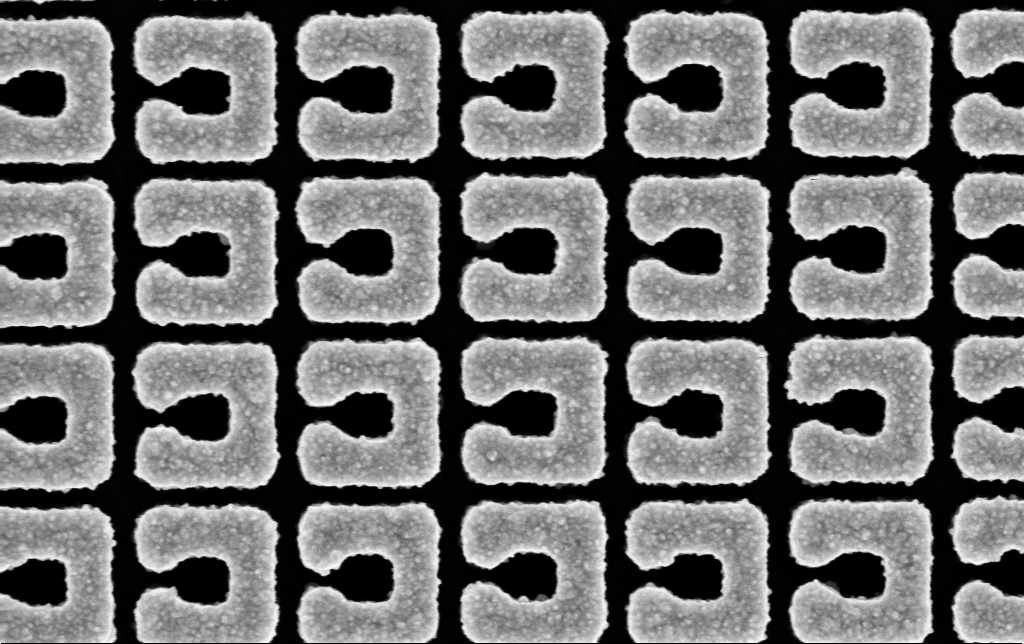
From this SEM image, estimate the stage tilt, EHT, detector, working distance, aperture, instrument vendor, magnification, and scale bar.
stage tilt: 0°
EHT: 5 kV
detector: InLens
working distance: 3.3 mm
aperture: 30 µm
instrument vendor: Zeiss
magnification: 131.52 K X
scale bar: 200 nm